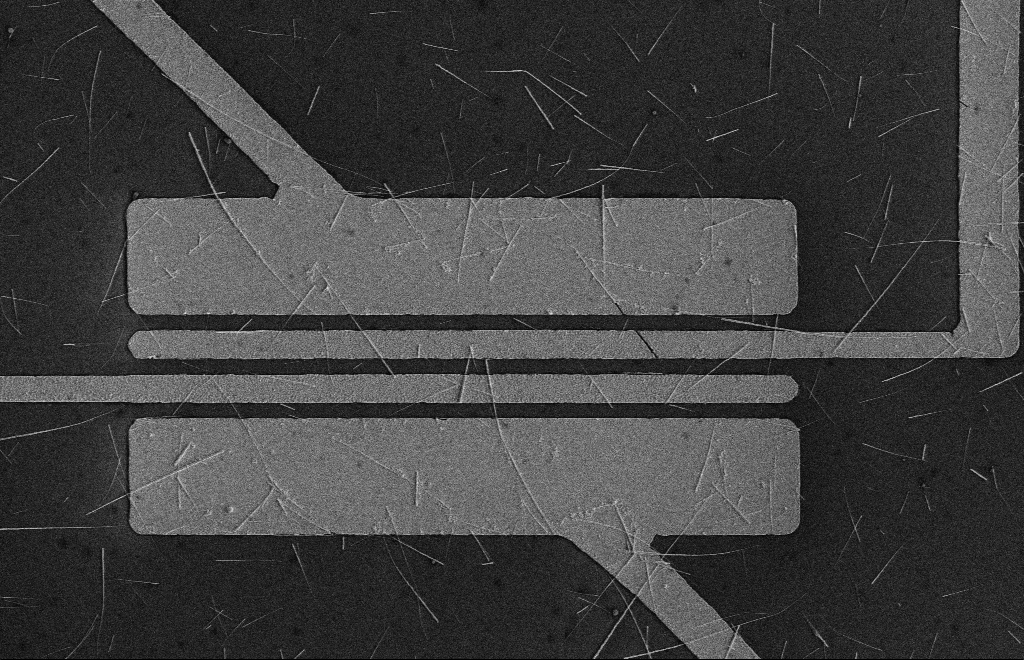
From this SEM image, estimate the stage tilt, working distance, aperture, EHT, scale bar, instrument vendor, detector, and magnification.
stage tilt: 0°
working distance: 16 mm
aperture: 10 µm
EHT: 5 kV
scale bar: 10000 nm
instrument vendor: Zeiss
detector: SE2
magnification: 4.08 K X